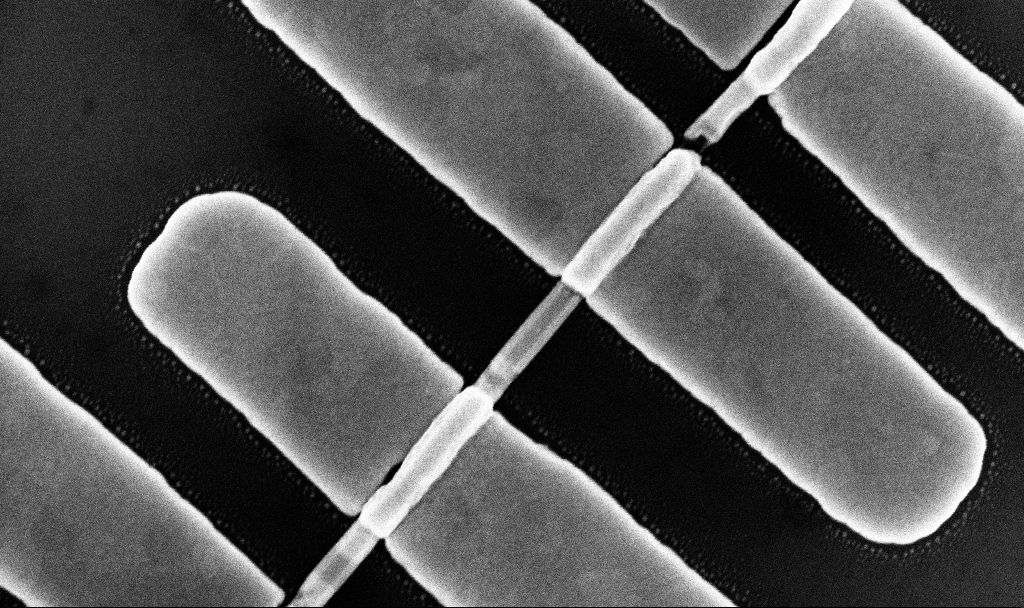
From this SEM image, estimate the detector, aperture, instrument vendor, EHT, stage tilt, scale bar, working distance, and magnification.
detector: InLens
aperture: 30 µm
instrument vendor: Zeiss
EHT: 10 kV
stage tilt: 0°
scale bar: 200 nm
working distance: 7.7 mm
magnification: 101.83 K X